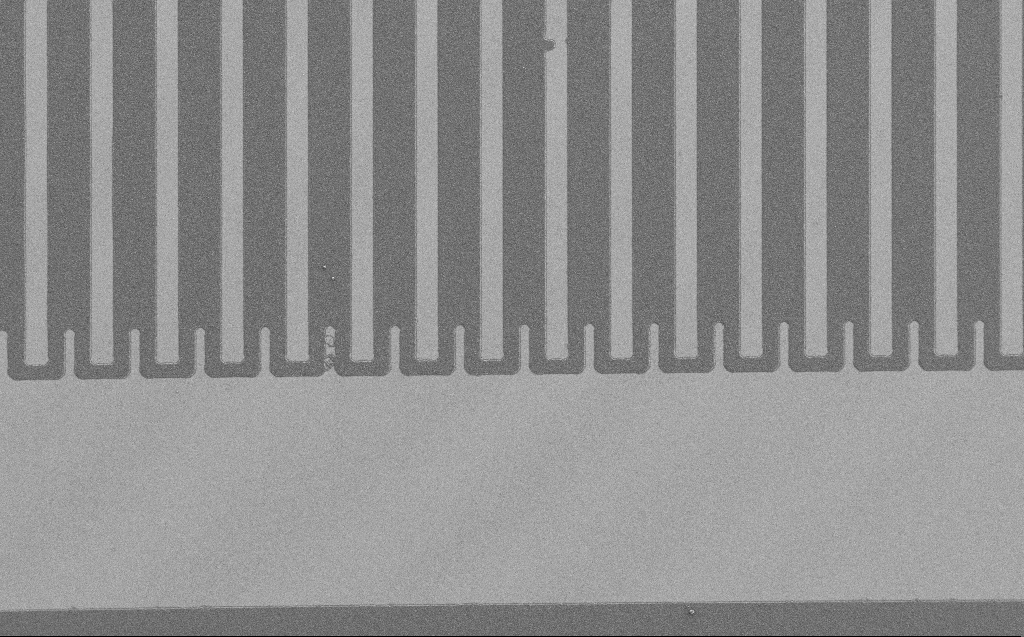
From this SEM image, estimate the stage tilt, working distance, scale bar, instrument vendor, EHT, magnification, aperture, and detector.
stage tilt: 0°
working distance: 4 mm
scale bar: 20000 nm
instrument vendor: Zeiss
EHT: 1.2 kV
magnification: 0.871 K X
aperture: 30 µm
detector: SE2